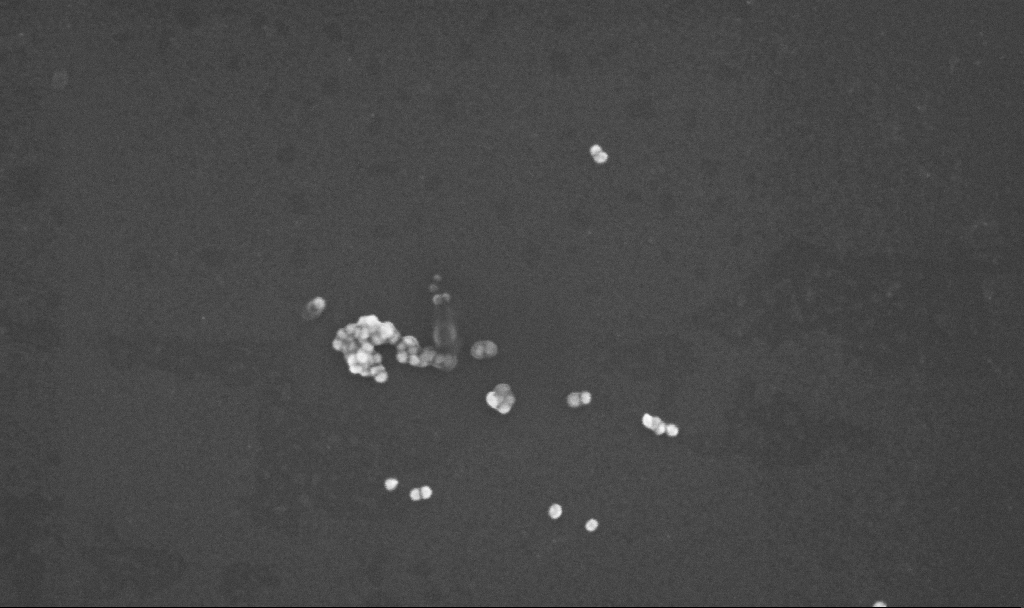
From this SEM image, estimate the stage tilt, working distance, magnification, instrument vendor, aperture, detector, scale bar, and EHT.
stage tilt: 0°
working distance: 3.2 mm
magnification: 200 K X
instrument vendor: Zeiss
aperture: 30 µm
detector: InLens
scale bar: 100 nm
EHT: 10 kV